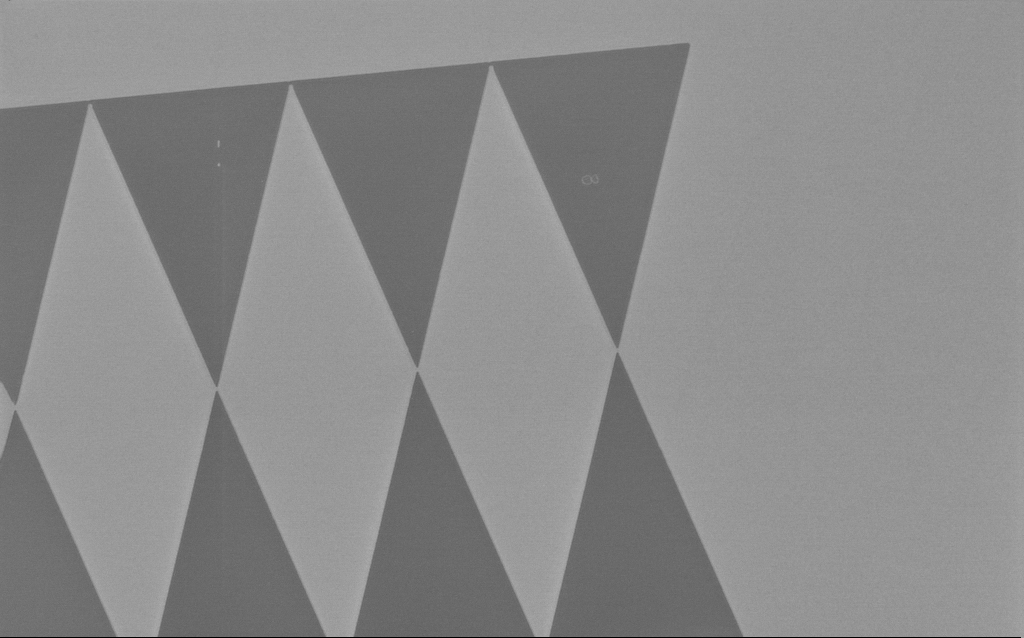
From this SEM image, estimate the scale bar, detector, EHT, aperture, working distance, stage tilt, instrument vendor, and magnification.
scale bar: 2000 nm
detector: InLens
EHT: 1 kV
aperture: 30 µm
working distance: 5 mm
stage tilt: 0°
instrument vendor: Zeiss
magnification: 7.34 K X